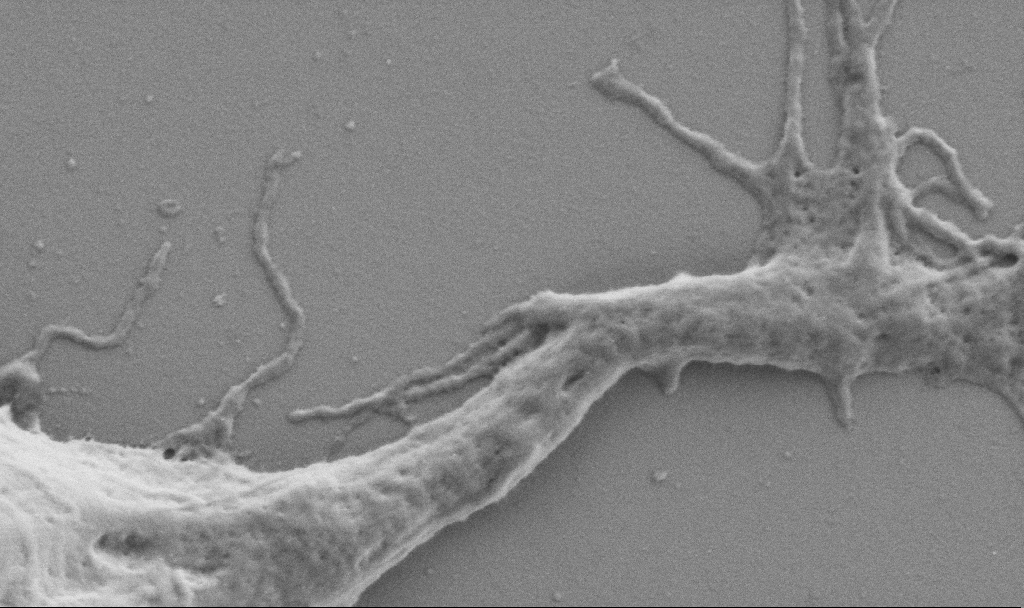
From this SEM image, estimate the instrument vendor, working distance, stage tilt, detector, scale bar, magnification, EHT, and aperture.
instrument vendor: Zeiss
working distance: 6.9 mm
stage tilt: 0°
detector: SE2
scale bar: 1000 nm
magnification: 50 K X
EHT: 0.9 kV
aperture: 30 µm